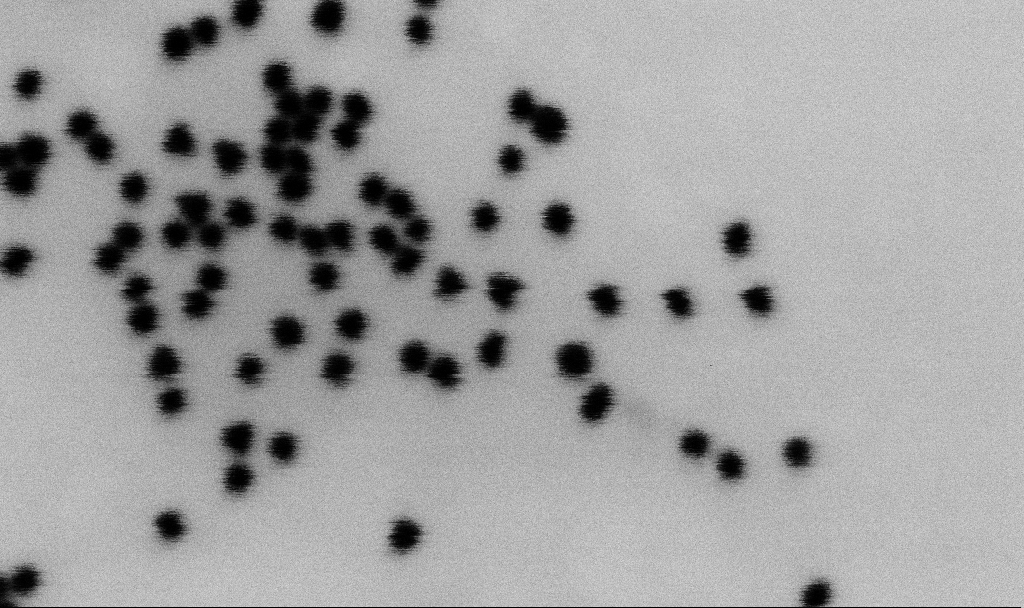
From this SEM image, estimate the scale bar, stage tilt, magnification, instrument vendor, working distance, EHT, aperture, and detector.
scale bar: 100 nm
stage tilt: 0°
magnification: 500 K X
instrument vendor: Zeiss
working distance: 6.5 mm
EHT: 2 kV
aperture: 30 µm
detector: SE2